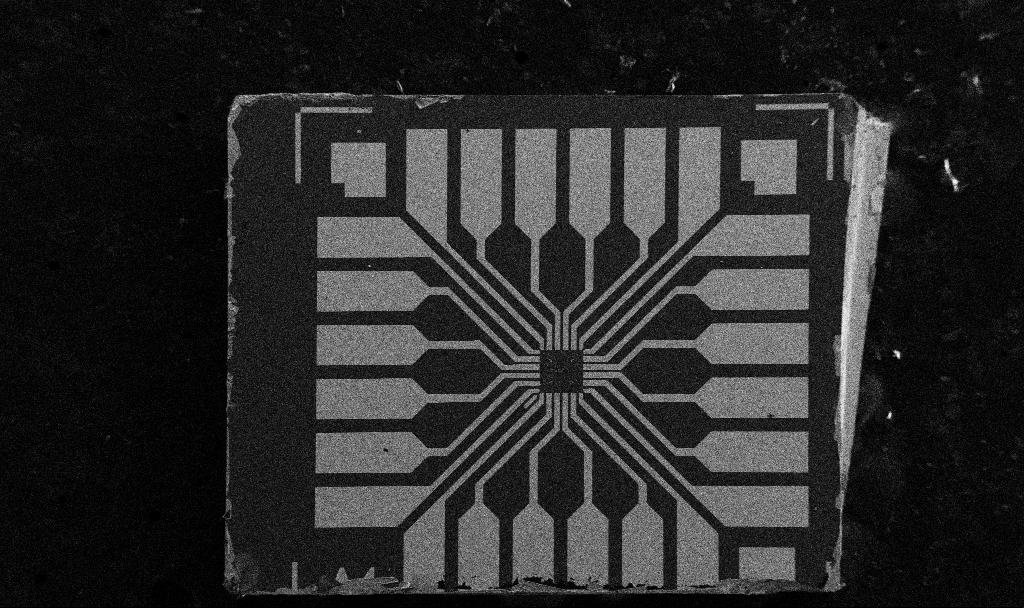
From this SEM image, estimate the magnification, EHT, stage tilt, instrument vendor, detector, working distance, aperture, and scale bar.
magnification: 0.1 K X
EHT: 5 kV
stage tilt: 0°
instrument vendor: Zeiss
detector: SE2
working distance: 10.7 mm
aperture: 30 µm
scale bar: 200000 nm